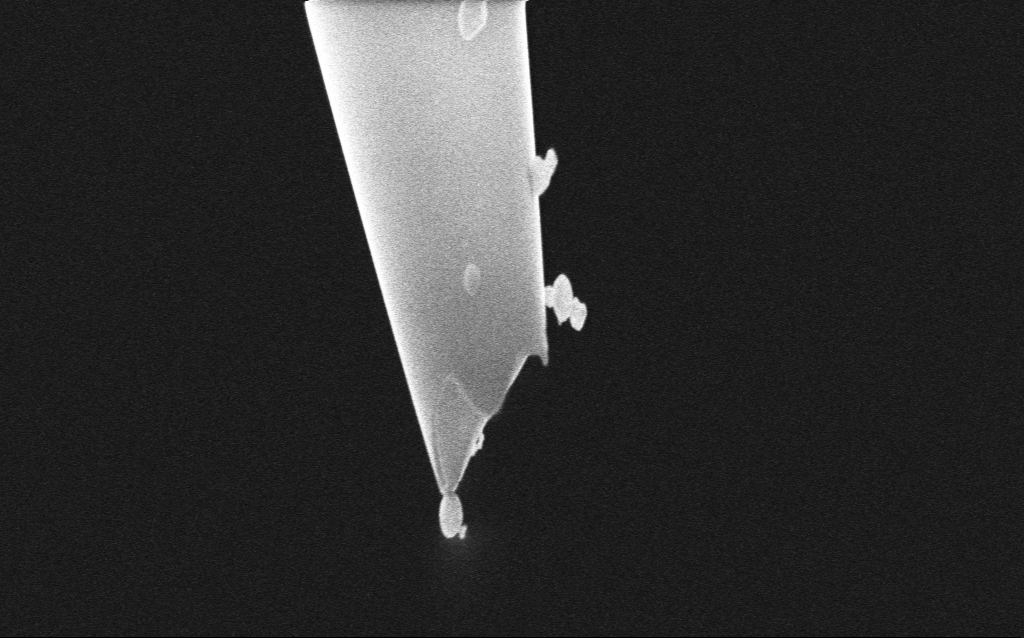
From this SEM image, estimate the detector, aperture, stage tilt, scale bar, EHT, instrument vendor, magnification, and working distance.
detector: InLens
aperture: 30 µm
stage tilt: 45°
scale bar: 1000 nm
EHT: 5 kV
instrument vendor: Zeiss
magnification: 47.87 K X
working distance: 3 mm